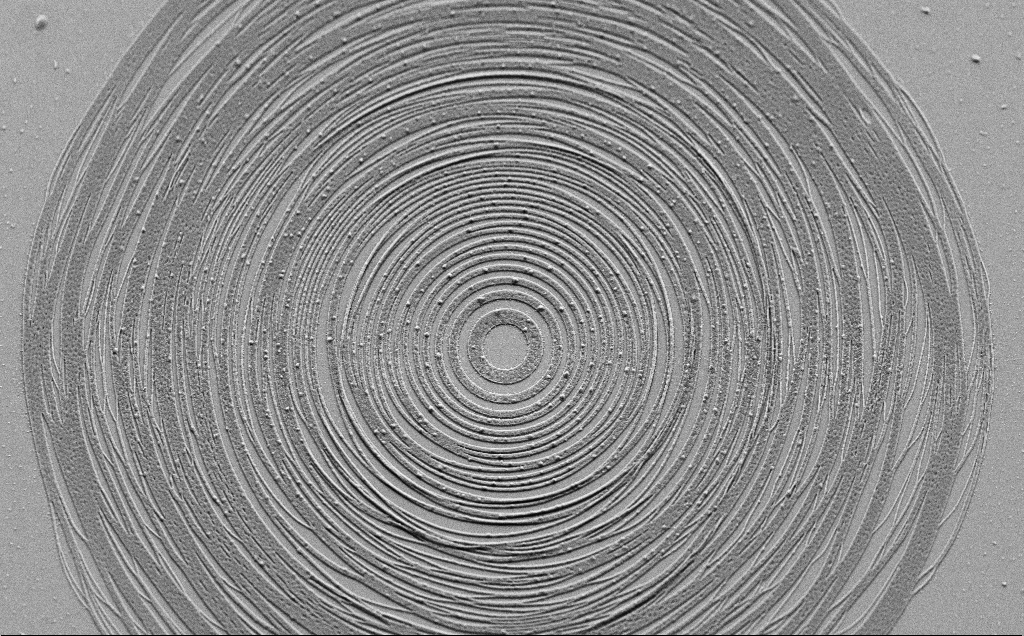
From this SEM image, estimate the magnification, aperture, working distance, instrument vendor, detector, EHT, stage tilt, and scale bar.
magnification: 2.29 K X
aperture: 30 µm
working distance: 6 mm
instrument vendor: Zeiss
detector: SE2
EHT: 5 kV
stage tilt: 45°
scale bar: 10000 nm